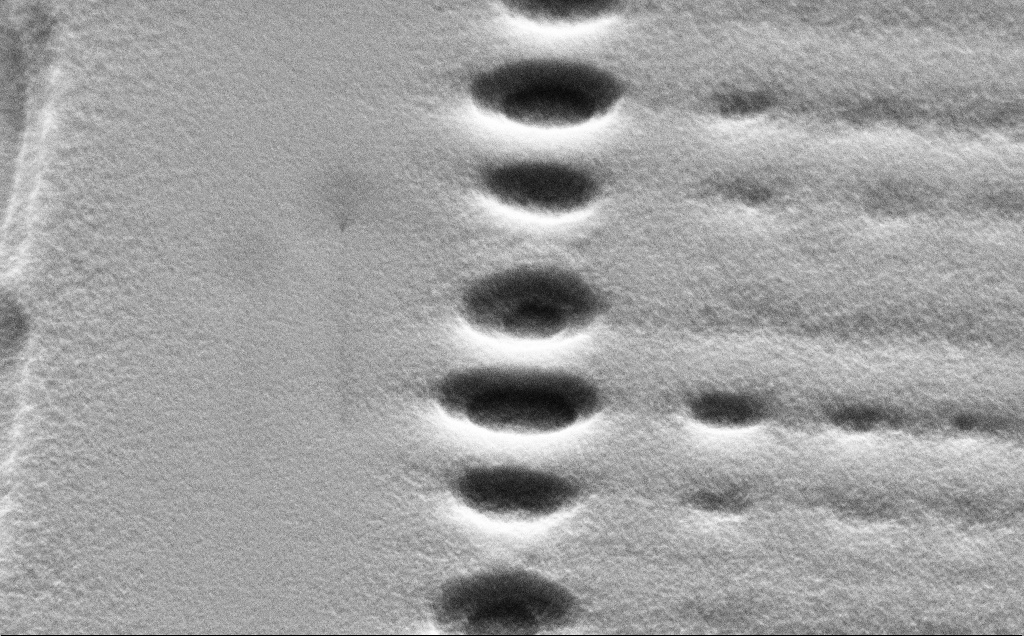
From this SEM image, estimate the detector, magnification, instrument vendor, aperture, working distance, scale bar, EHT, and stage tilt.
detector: SE2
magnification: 28.65 K X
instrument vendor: Zeiss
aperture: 30 µm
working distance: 9 mm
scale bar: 2000 nm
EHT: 5 kV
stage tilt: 45°